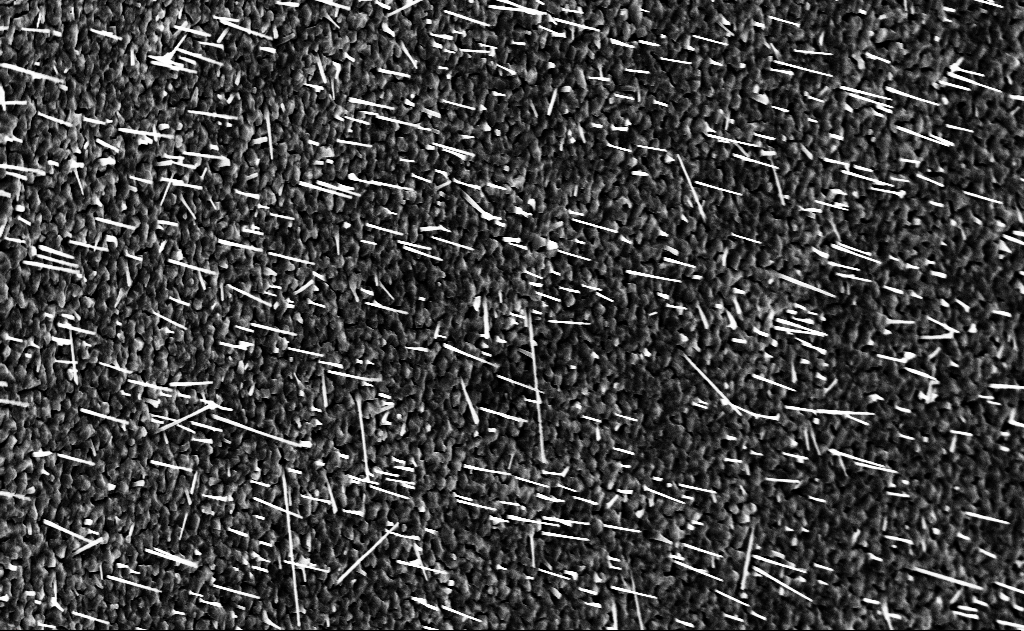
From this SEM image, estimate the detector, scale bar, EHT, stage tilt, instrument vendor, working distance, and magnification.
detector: InLens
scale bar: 2000 nm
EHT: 10 kV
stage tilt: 0°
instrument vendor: Zeiss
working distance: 14 mm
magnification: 10 K X